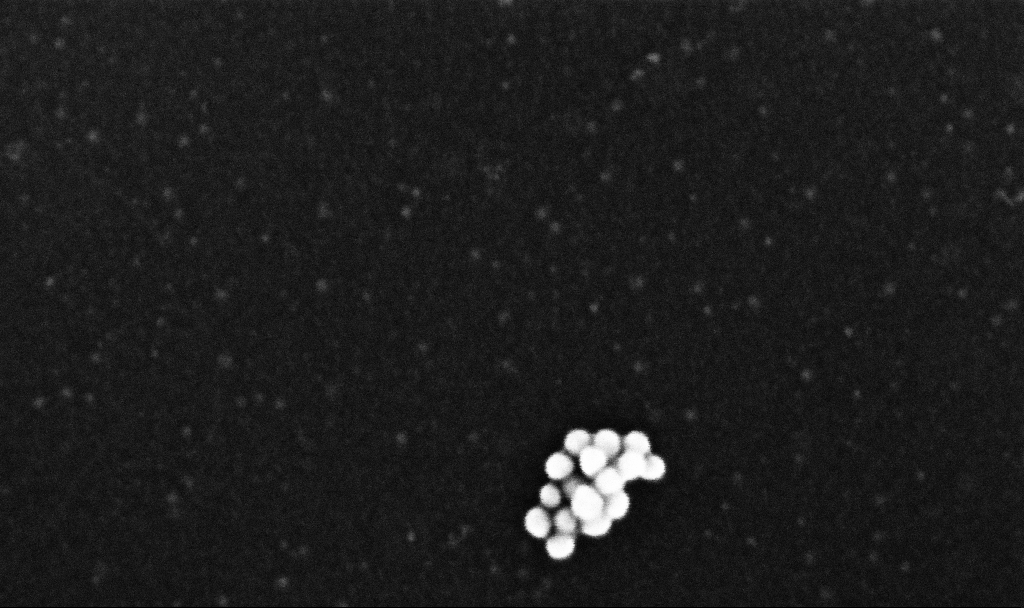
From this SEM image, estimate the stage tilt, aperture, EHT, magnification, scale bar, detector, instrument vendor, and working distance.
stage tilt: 0°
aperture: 30 µm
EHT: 10 kV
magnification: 459.48 K X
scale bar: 100 nm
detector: InLens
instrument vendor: Zeiss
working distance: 3.2 mm